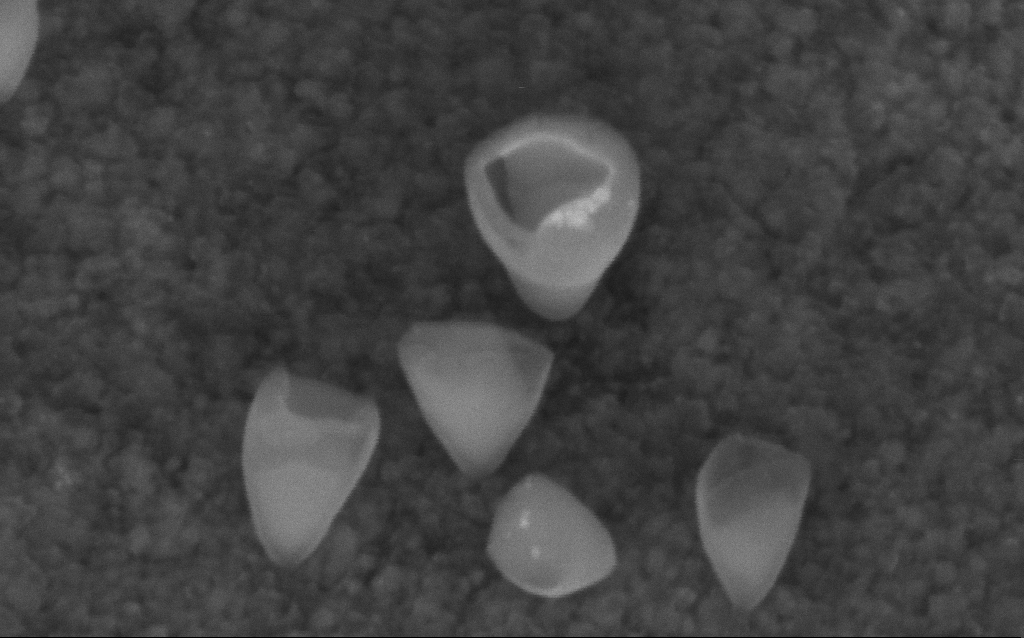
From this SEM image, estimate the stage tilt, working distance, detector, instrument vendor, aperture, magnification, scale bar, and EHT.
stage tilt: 45°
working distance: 7 mm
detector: InLens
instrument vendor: Zeiss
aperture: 30 µm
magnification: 416.83 K X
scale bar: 100 nm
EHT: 5 kV